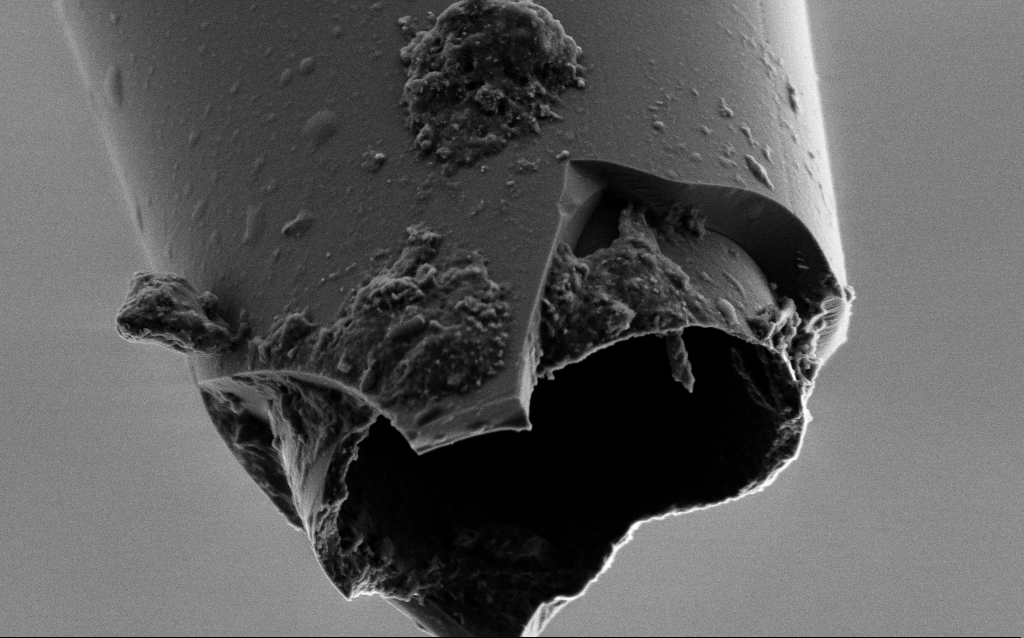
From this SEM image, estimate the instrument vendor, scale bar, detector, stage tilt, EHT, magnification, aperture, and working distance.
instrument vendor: Zeiss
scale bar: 1000 nm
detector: SE2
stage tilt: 45°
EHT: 2 kV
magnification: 25 K X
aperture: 30 µm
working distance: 6 mm